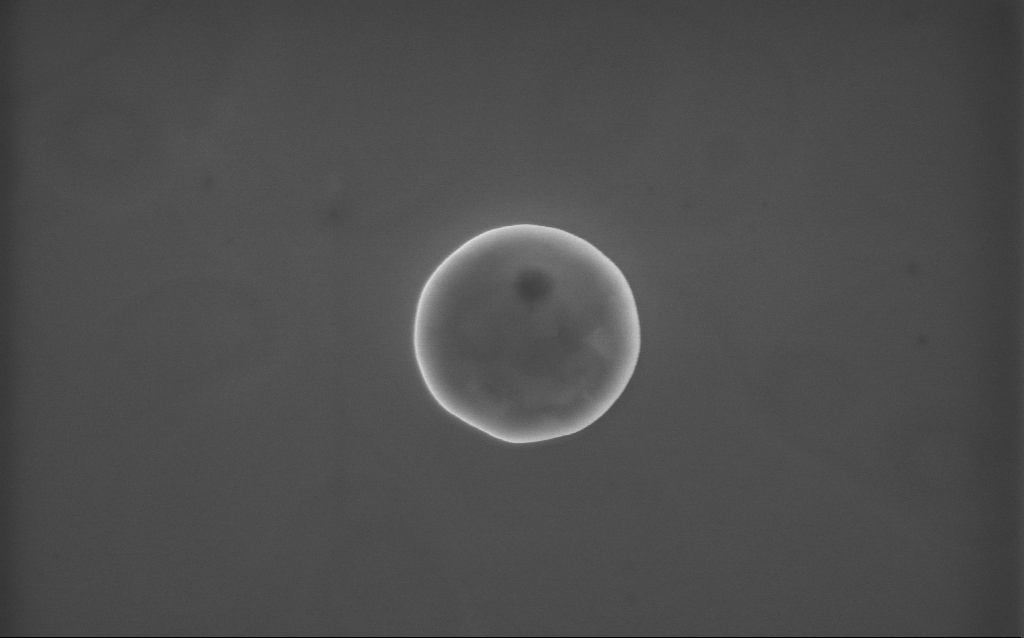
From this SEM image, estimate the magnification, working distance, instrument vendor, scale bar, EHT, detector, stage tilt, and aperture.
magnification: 65 K X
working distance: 2 mm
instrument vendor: Zeiss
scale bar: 1000 nm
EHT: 10 kV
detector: InLens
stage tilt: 0°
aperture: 30 µm